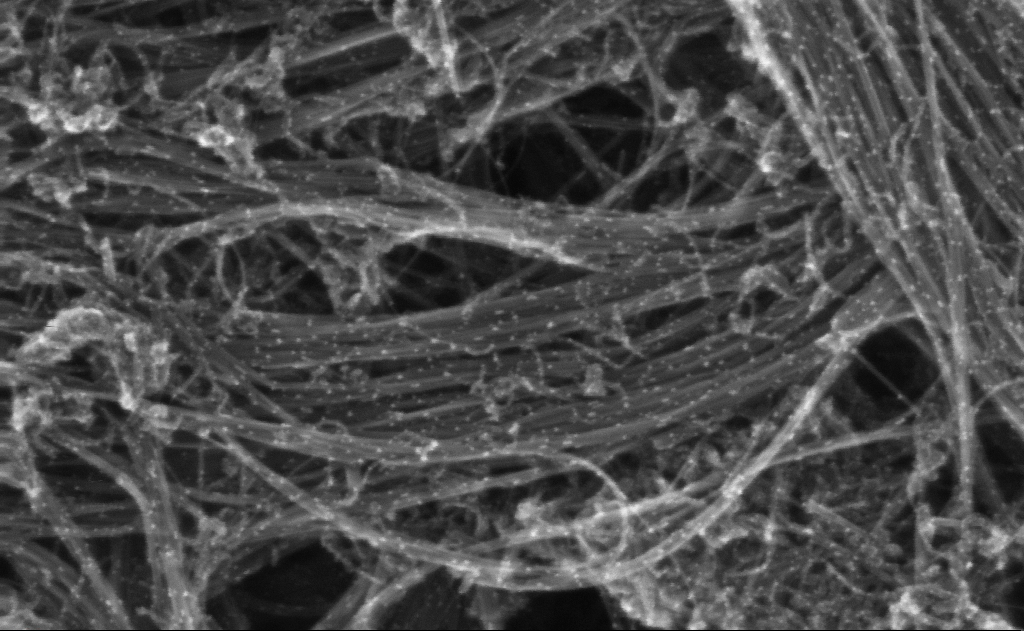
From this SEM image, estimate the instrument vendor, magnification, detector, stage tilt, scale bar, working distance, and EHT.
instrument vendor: Zeiss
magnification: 471.11 K X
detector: InLens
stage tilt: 0°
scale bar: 100 nm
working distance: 3 mm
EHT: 10 kV